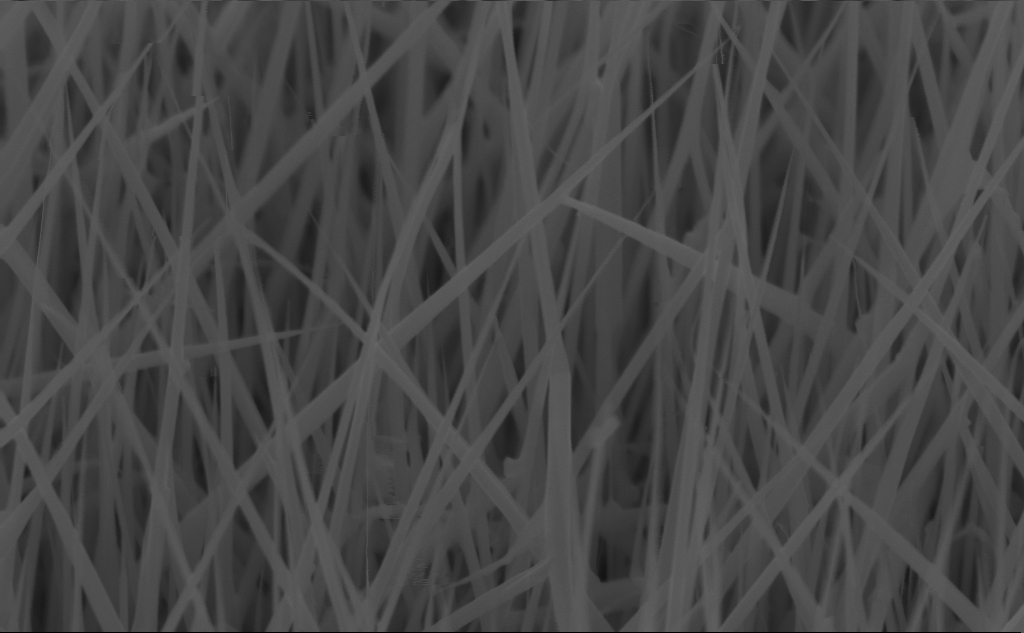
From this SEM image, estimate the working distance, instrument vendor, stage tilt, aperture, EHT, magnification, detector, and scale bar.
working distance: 4 mm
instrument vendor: Zeiss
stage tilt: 45°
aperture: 30 µm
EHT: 10 kV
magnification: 80 K X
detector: InLens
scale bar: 200 nm